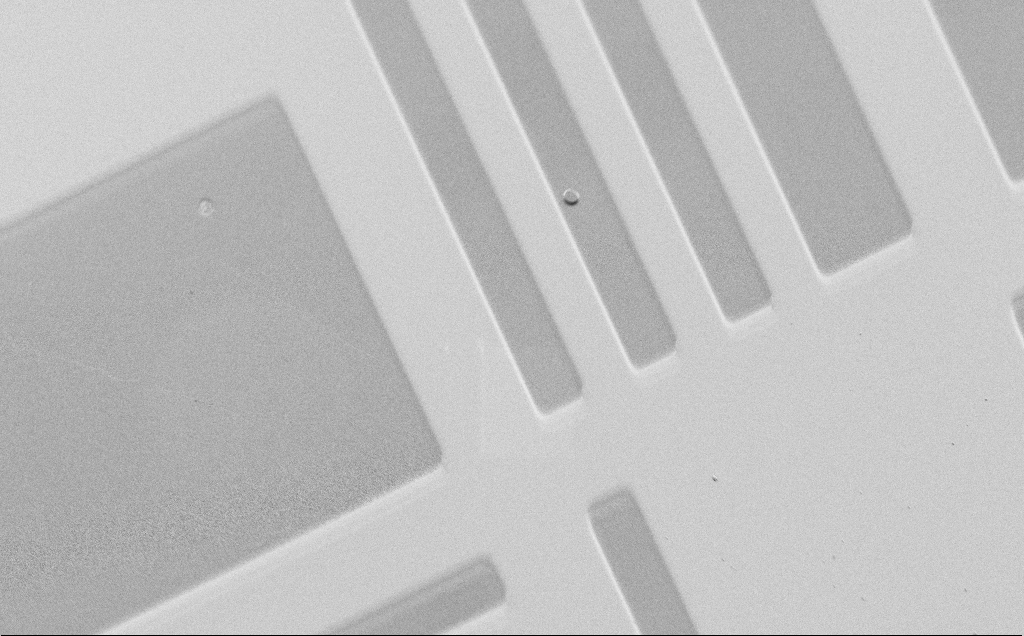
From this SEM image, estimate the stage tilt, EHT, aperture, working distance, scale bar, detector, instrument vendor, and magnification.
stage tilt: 45°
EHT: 1.5 kV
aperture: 30 µm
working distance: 4 mm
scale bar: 20000 nm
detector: SE2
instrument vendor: Zeiss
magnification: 0.766 K X